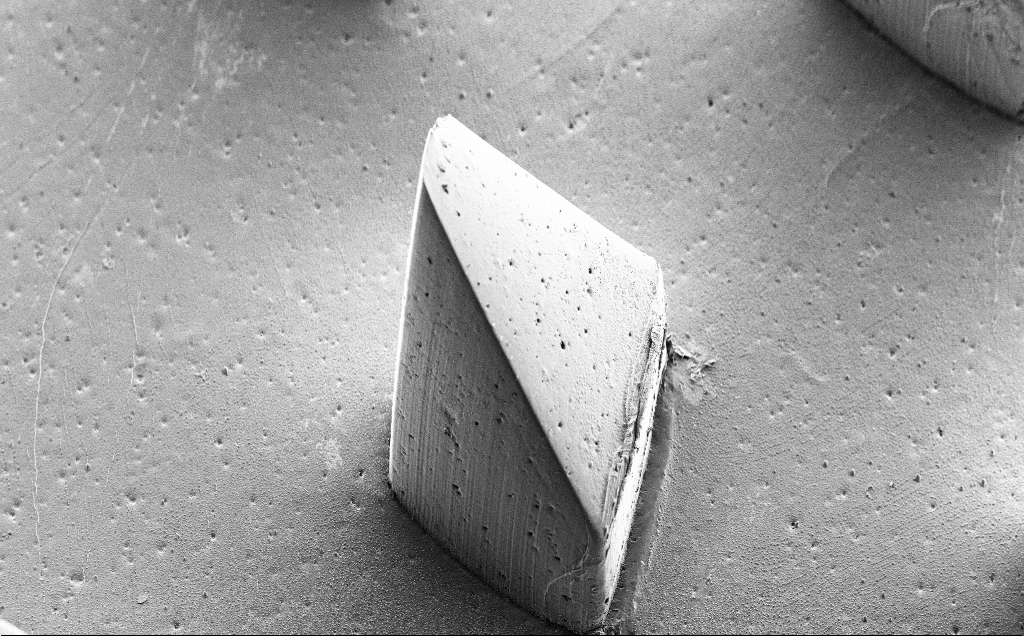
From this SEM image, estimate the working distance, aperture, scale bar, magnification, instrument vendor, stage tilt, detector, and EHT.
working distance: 8 mm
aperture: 30 µm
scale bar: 200000 nm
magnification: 0.263 K X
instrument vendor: Zeiss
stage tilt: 39°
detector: SE2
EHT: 5 kV